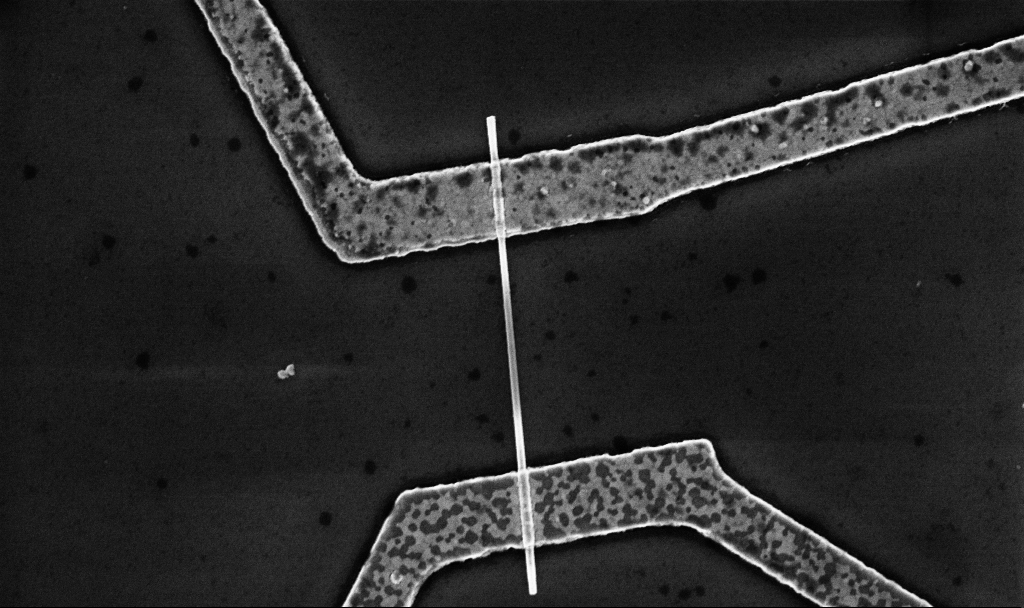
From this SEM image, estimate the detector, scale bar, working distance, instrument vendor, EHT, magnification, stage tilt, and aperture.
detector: InLens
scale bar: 1000 nm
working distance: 8.7 mm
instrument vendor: Zeiss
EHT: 5 kV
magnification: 30 K X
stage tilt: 0°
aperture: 30 µm